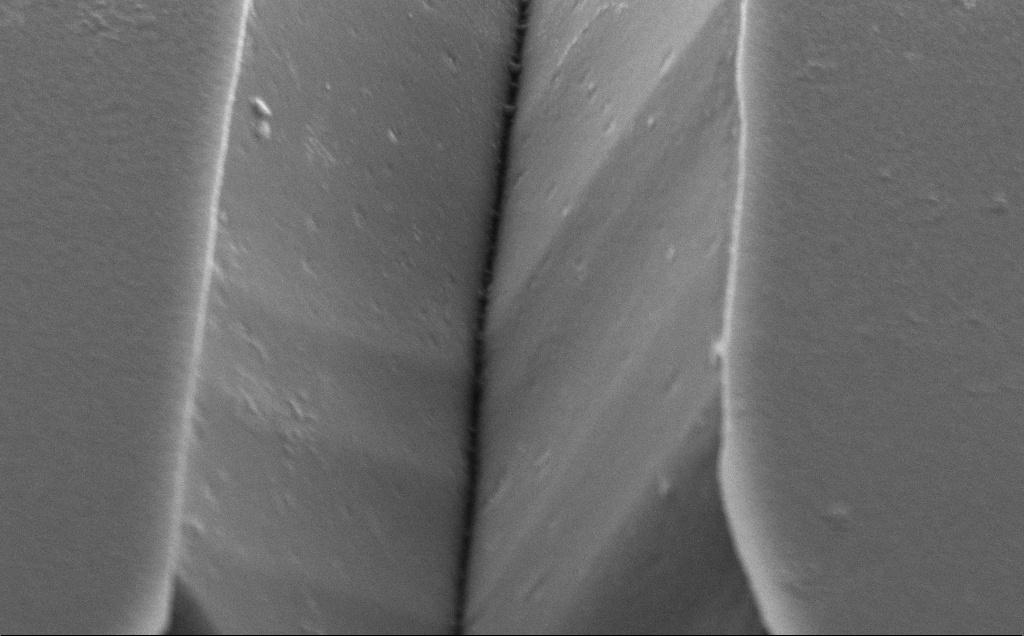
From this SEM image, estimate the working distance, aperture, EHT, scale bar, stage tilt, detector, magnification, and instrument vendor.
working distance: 10 mm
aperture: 30 µm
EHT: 5 kV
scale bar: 1000 nm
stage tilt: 50°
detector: SE2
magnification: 60.3 K X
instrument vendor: Zeiss